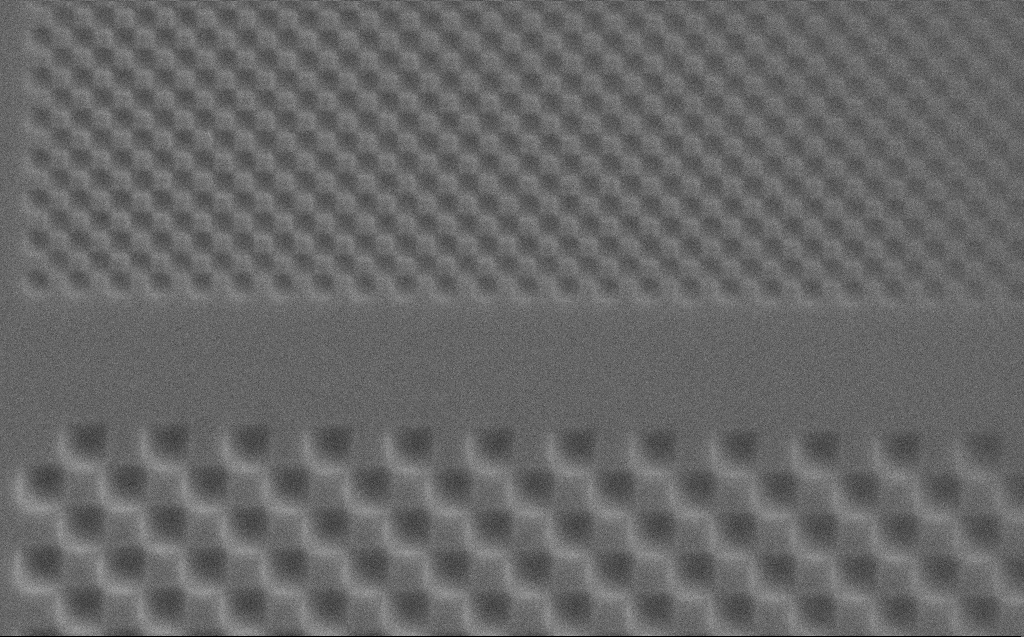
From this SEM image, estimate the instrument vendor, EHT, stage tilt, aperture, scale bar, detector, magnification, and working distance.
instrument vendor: Zeiss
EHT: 5 kV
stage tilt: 0°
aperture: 30 µm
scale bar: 2000 nm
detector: SE2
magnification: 15.12 K X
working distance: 4 mm